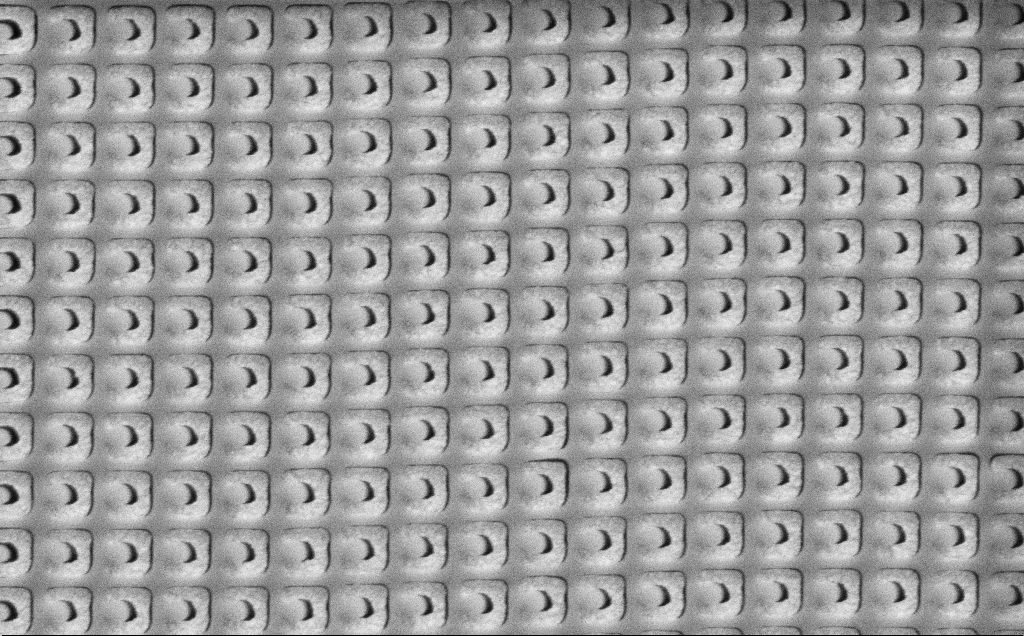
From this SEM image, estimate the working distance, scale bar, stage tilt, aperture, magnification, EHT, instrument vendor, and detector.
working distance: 8.3 mm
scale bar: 1000 nm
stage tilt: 0°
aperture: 30 µm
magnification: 46.34 K X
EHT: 3 kV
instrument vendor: Zeiss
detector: SE2